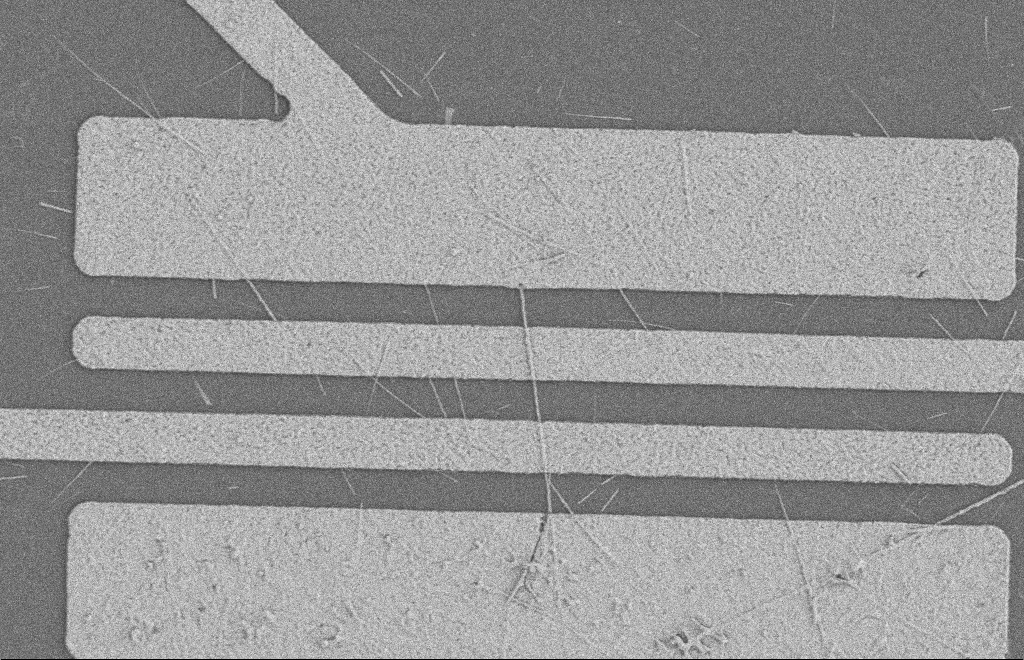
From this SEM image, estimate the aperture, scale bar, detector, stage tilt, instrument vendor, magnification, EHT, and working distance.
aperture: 20 µm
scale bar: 2000 nm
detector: SE2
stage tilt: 0°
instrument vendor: Zeiss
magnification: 5.67 K X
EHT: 2 kV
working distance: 8 mm